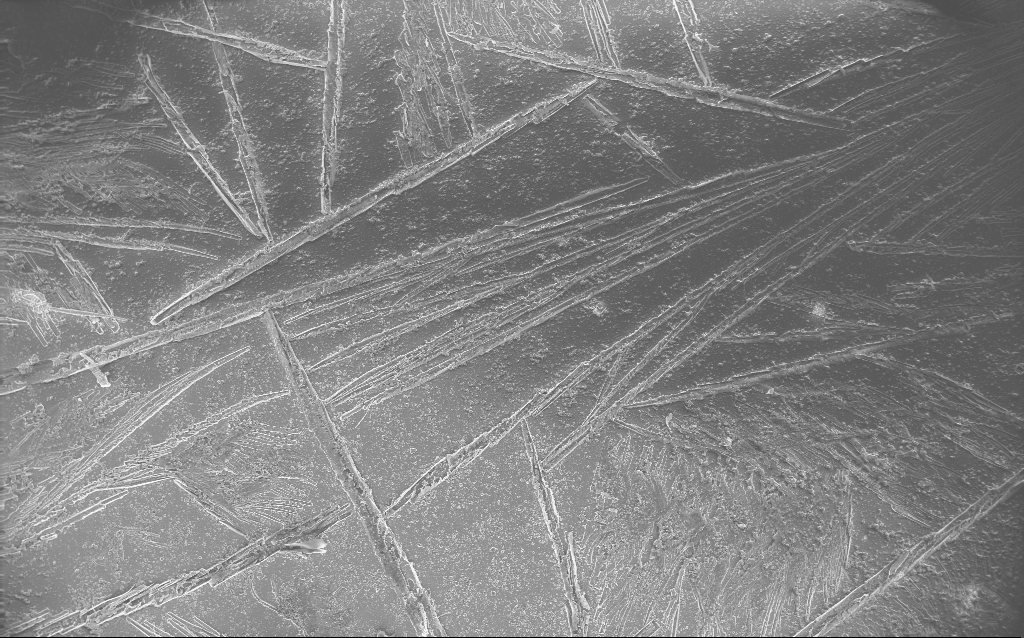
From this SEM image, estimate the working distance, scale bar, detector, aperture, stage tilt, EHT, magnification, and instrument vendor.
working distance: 2.5 mm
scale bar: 100000 nm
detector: InLens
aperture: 30 µm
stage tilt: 0°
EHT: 10 kV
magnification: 0.125 K X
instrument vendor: Zeiss